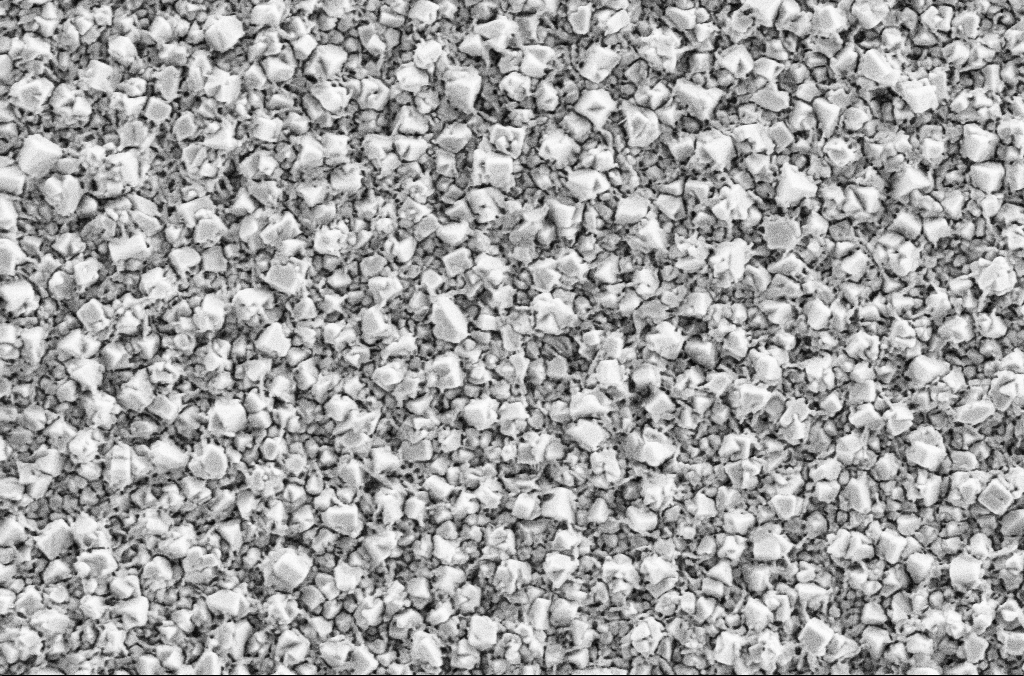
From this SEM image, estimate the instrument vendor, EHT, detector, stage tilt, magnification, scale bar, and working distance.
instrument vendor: Zeiss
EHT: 2 kV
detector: SE2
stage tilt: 0°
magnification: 30 K X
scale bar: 1000 nm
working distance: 1.9 mm